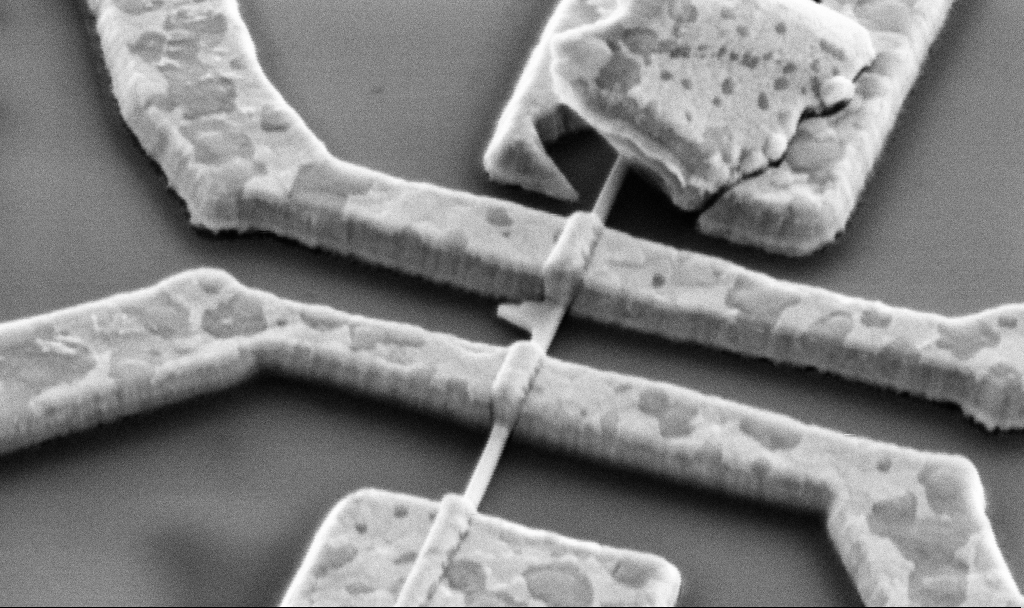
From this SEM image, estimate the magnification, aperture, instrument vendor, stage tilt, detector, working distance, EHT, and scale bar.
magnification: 60 K X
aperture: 30 µm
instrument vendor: Zeiss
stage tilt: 45°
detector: SE2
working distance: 14.7 mm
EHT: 5 kV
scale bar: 1000 nm